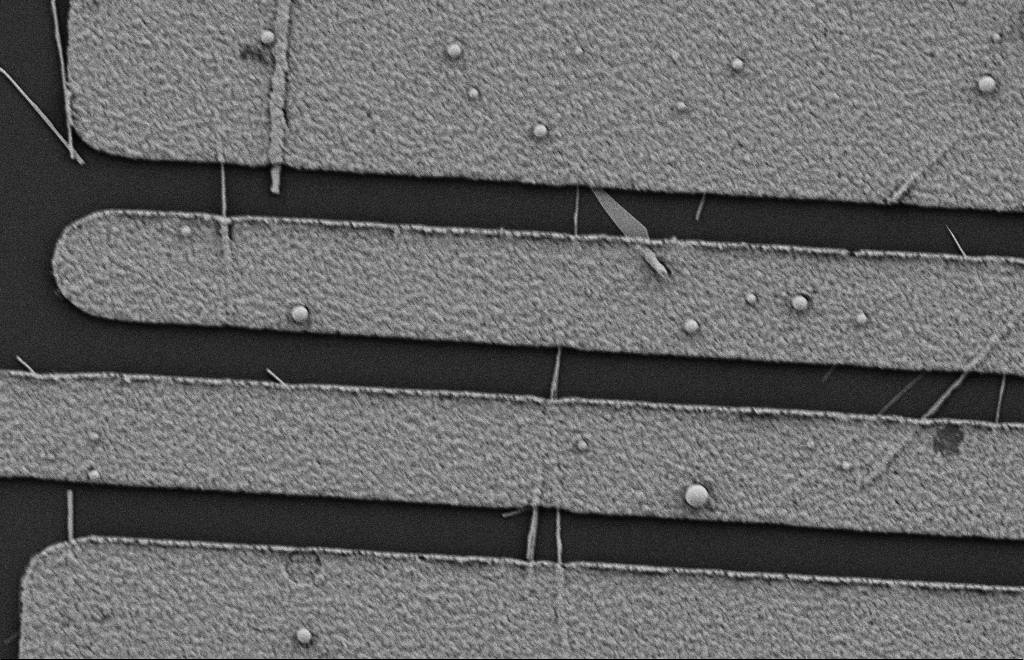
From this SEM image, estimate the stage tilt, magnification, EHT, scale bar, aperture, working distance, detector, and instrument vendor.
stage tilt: -0.3°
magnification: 15 K X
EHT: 2 kV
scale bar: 2000 nm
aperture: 20 µm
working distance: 10 mm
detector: SE2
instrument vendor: Zeiss